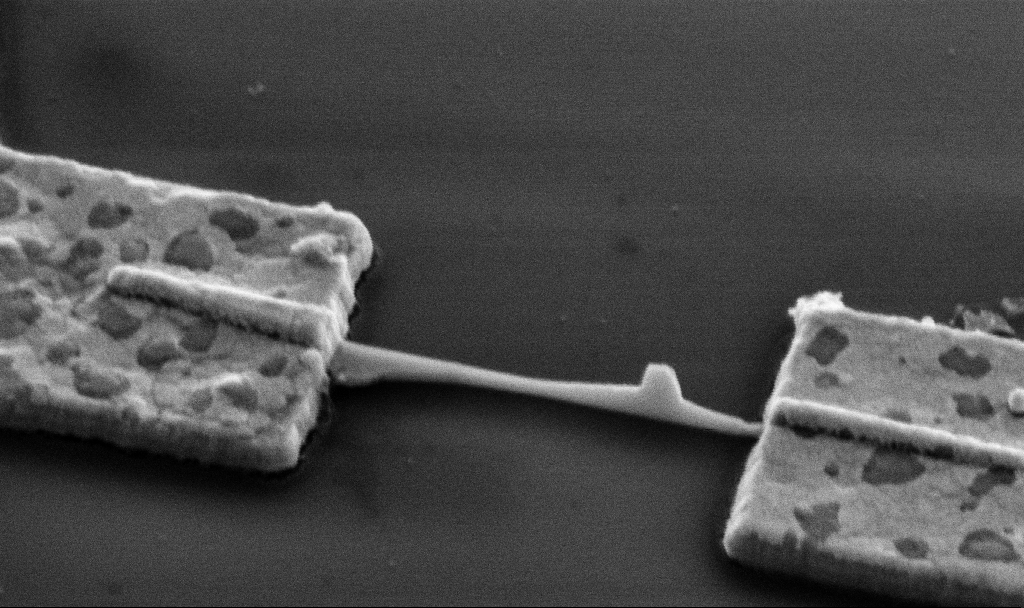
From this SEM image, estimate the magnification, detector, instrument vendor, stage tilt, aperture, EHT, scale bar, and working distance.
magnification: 60 K X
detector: SE2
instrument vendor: Zeiss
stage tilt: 45°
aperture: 30 µm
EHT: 5 kV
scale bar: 1000 nm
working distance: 13.6 mm